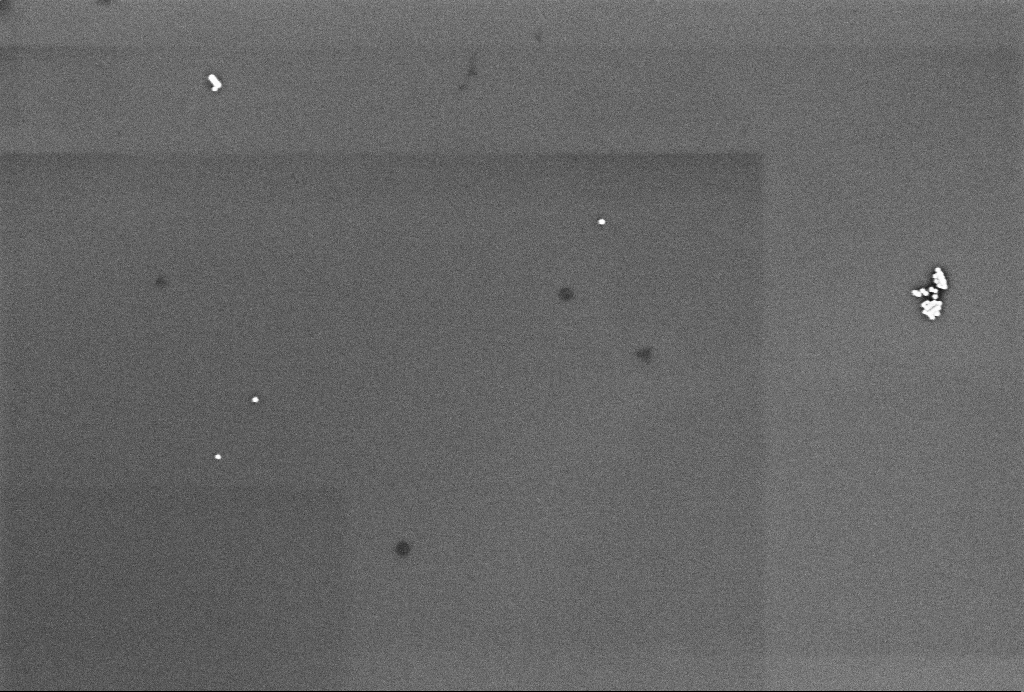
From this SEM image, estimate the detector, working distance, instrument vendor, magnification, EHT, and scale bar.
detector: InLens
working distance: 3.3 mm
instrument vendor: Zeiss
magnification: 74.02 K X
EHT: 2 kV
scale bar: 200 nm